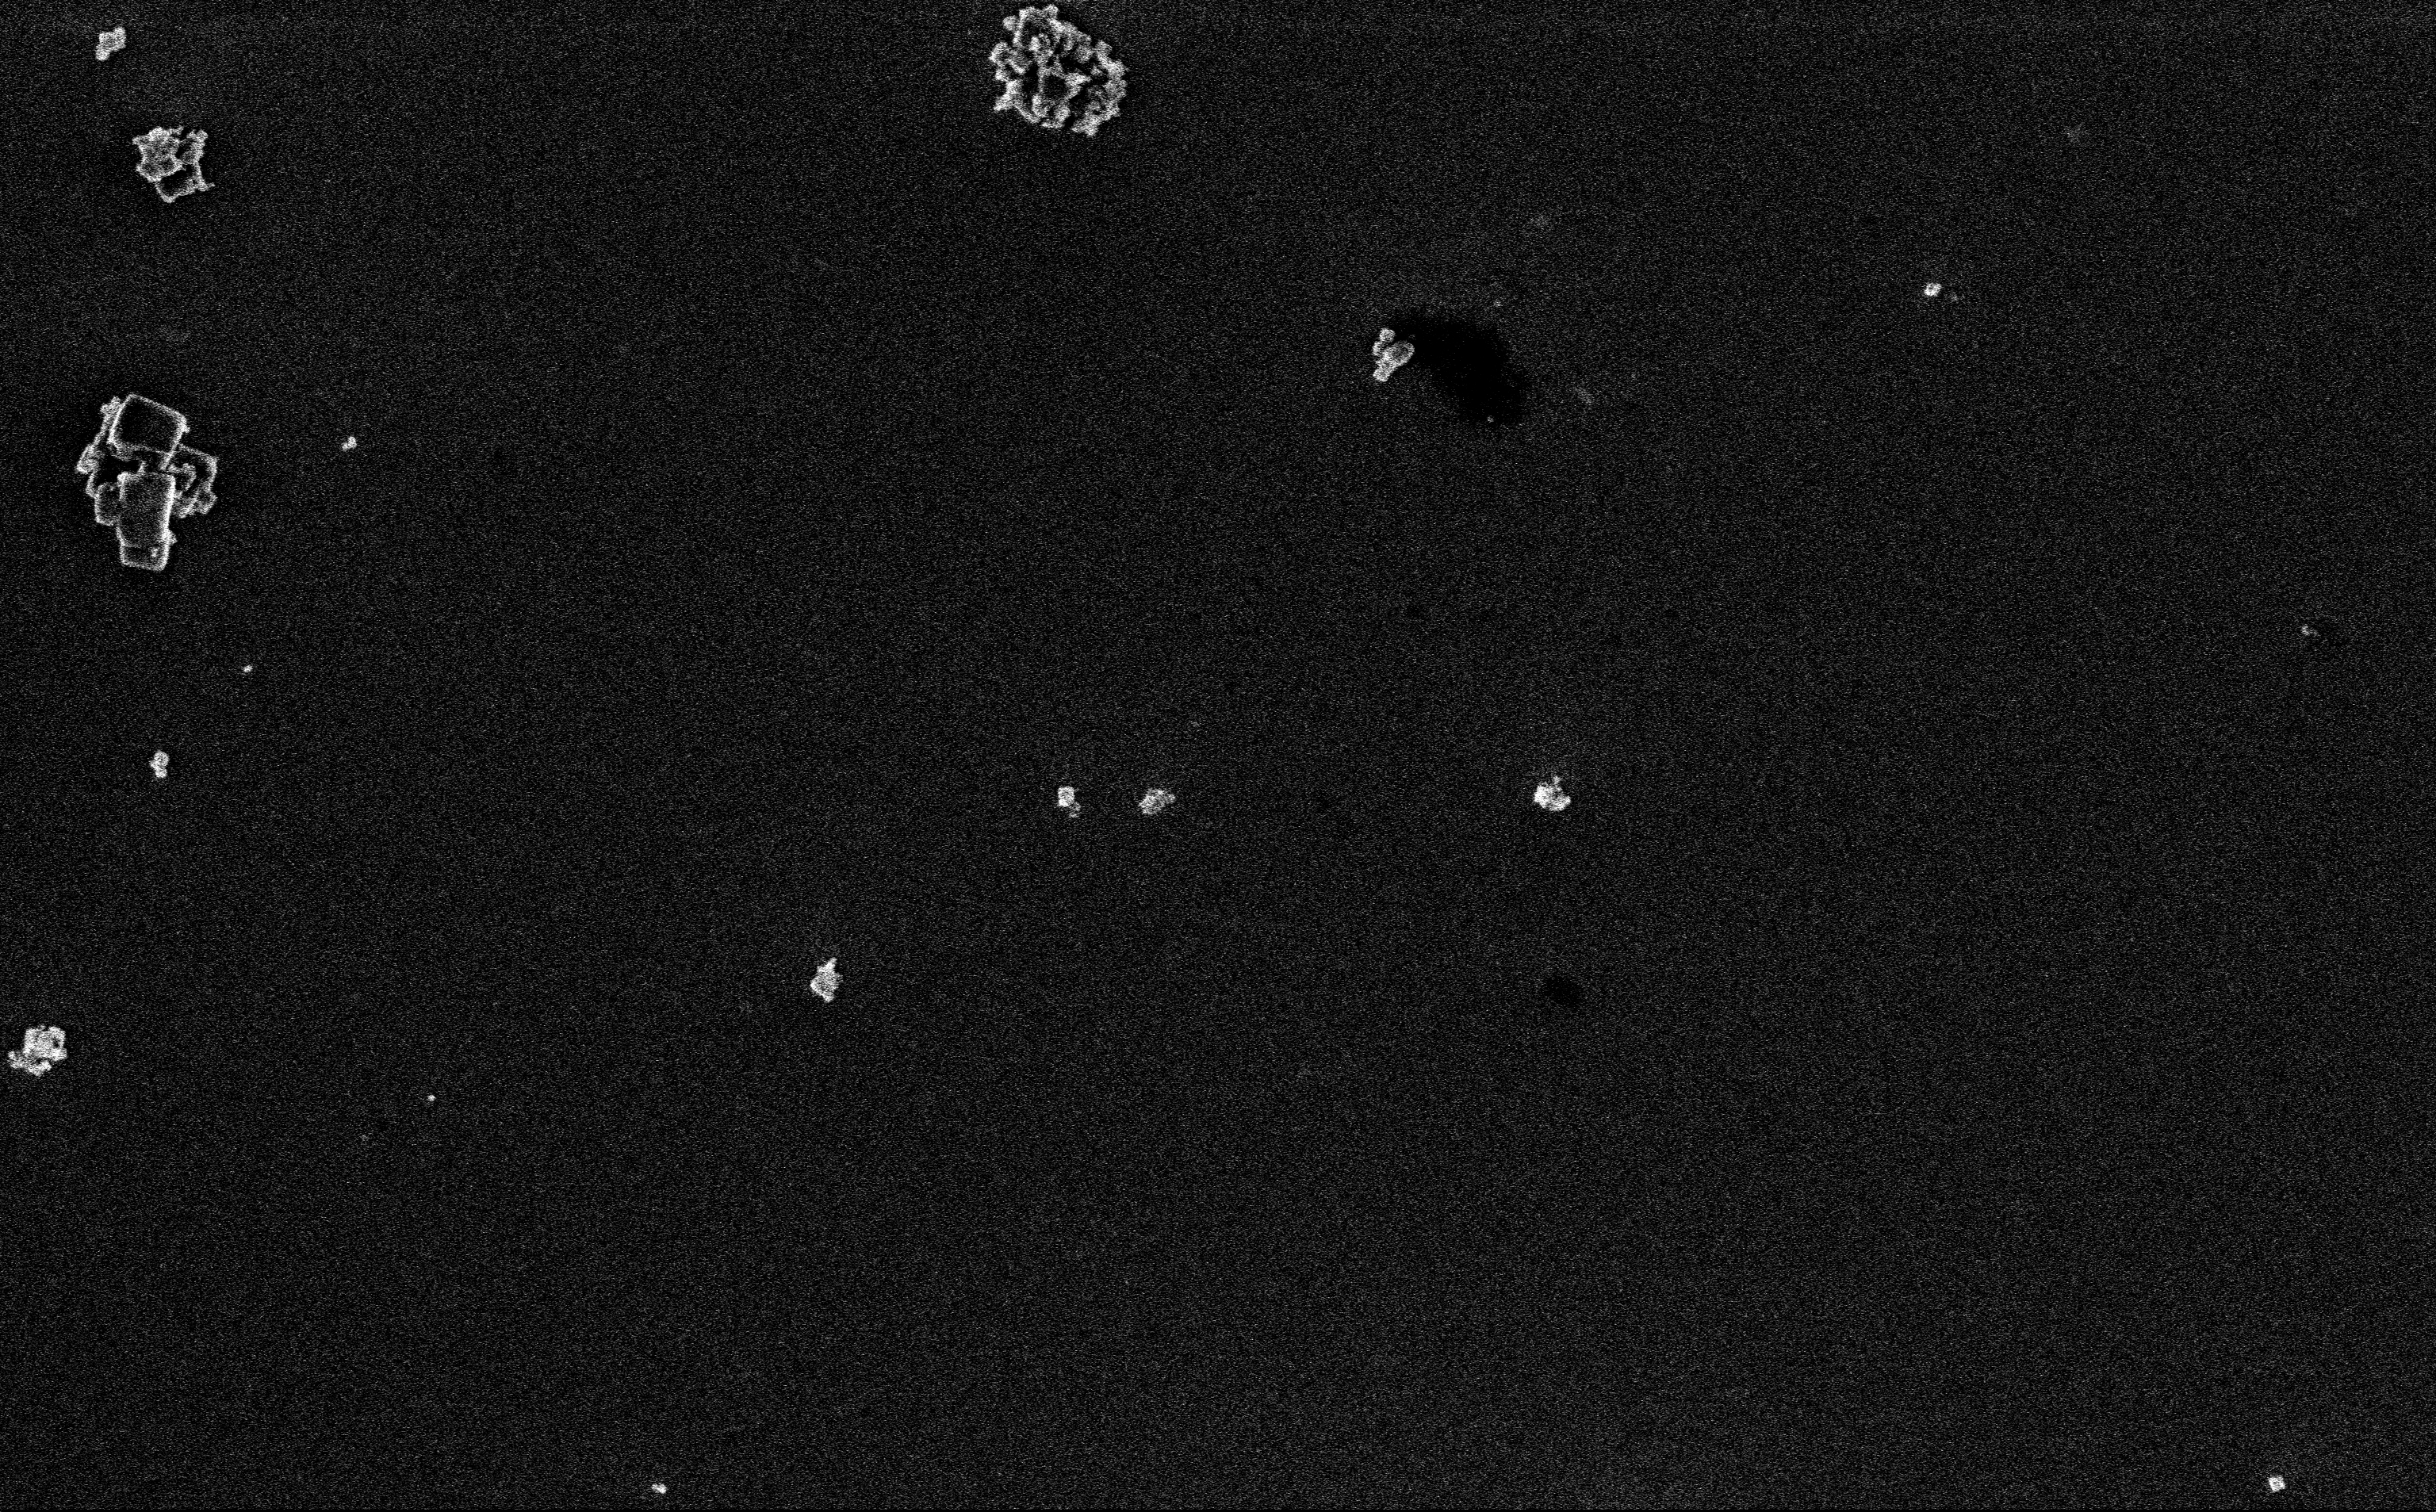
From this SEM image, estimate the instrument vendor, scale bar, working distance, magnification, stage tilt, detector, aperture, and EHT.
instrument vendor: Zeiss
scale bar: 2000 nm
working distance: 3 mm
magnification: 12.85 K X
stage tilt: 0°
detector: InLens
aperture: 30 µm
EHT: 3 kV